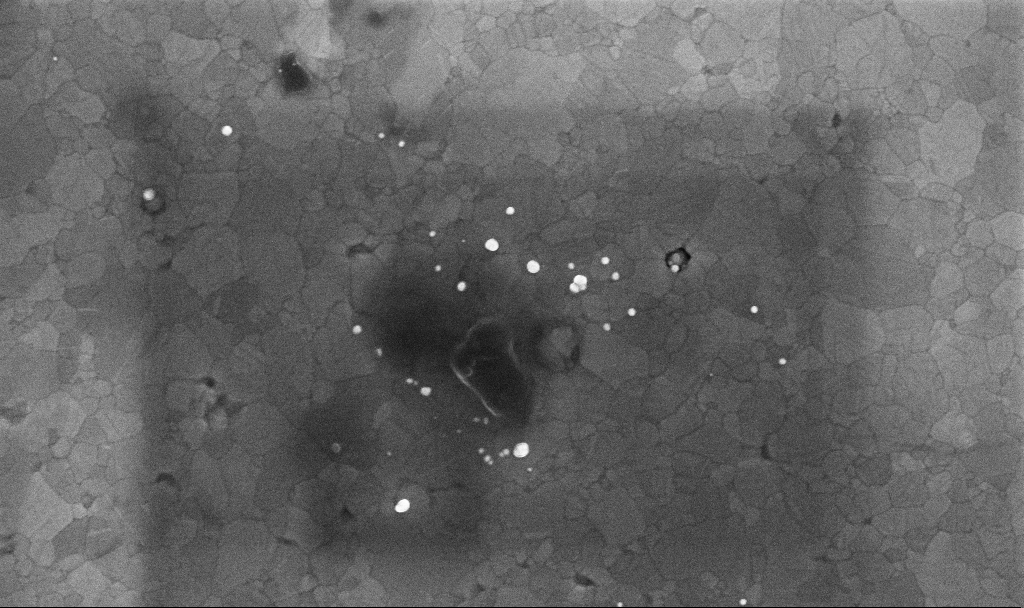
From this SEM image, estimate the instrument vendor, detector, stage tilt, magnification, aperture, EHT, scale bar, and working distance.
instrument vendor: Zeiss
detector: InLens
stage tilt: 0°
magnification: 70.32 K X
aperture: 30 µm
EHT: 10 kV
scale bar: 1000 nm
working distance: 3.4 mm